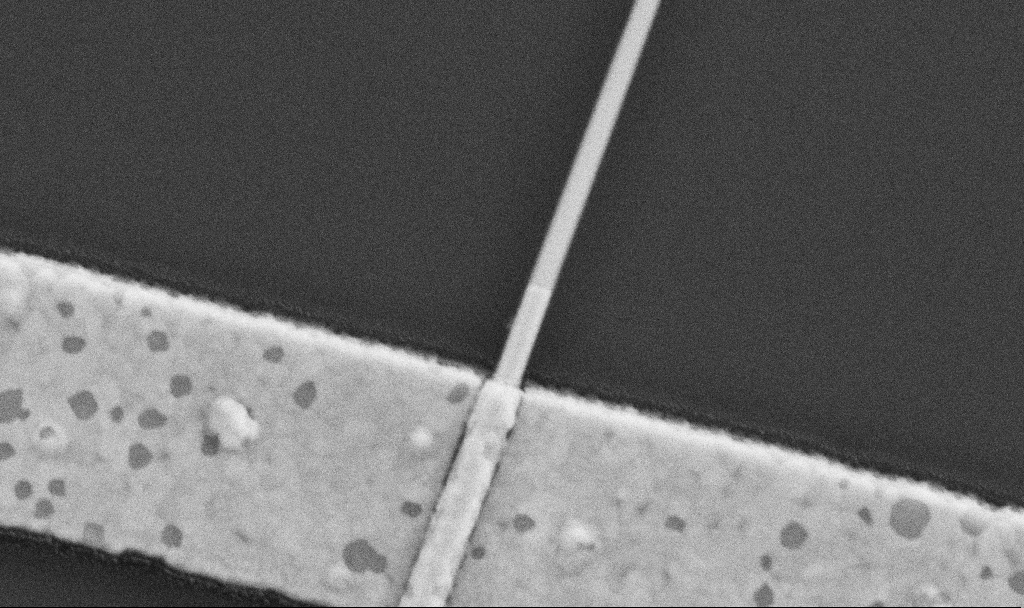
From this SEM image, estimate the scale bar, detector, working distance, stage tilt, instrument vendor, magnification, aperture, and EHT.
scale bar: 200 nm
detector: SE2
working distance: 8.7 mm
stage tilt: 0°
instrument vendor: Zeiss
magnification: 100 K X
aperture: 30 µm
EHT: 5 kV